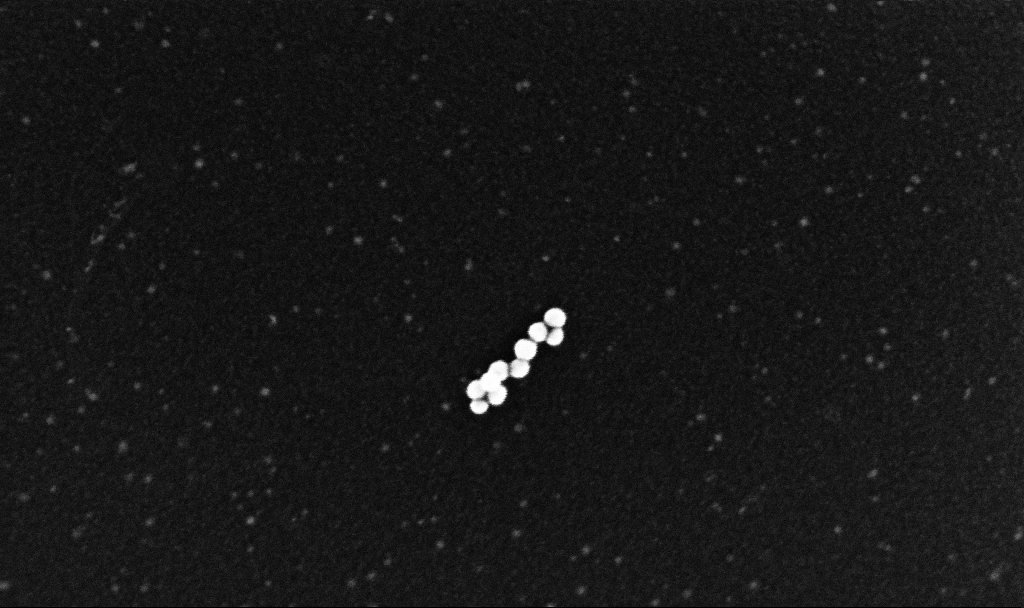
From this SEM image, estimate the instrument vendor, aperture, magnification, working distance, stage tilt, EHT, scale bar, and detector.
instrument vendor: Zeiss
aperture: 30 µm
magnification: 316.26 K X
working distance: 3.2 mm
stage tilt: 0°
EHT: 10 kV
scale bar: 200 nm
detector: InLens